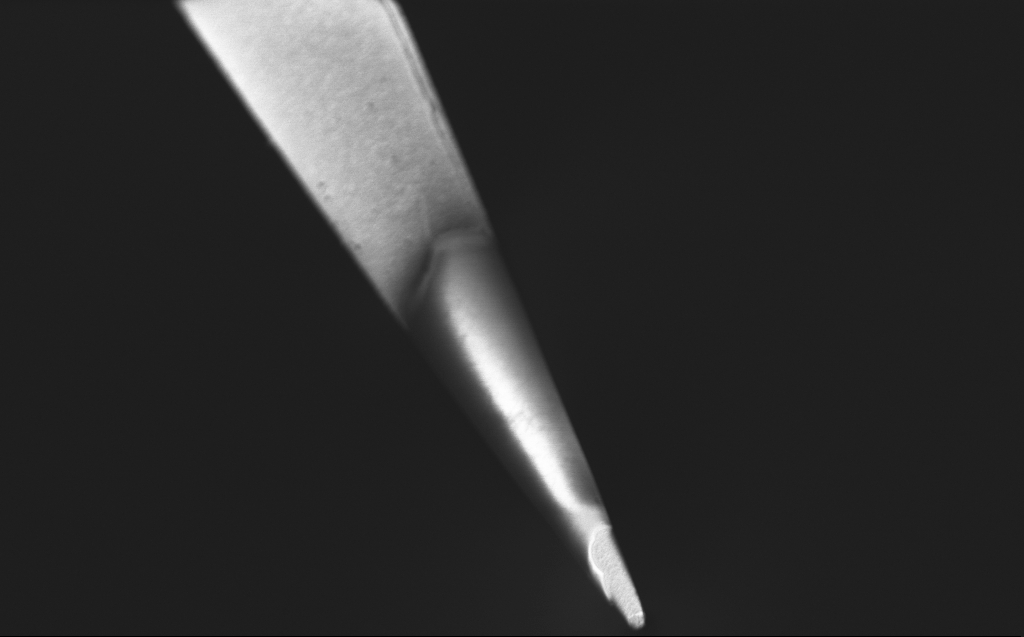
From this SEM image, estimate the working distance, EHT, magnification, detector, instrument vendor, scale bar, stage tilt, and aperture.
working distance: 4 mm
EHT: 0.8 kV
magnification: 18.21 K X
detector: InLens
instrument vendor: Zeiss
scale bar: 2000 nm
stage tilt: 45°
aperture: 30 µm